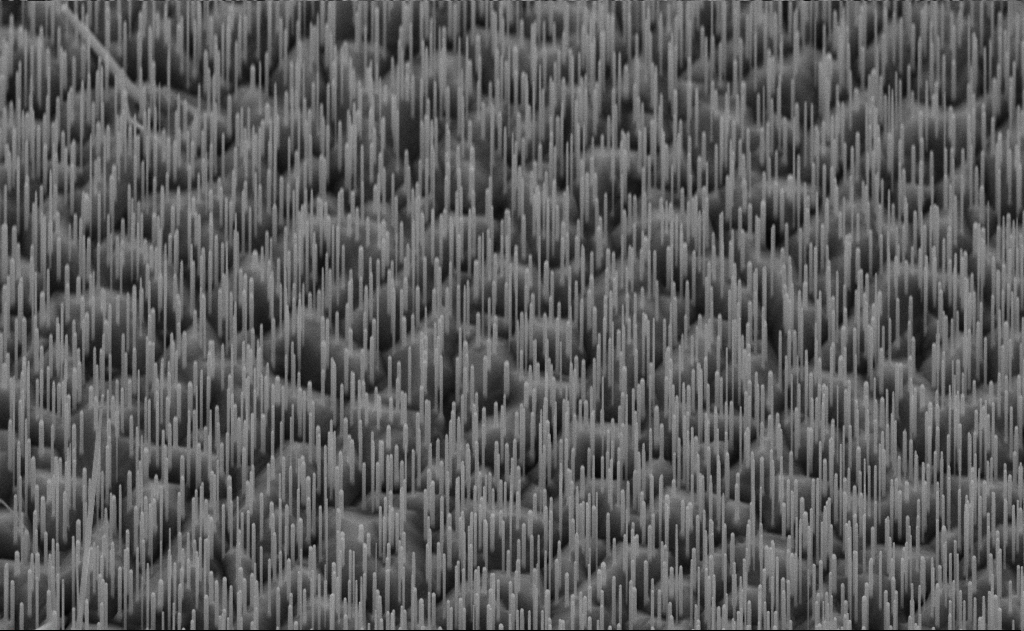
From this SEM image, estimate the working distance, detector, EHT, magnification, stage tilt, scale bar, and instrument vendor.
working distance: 10 mm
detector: SE2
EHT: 10 kV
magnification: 20 K X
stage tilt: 45°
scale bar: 2000 nm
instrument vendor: Zeiss